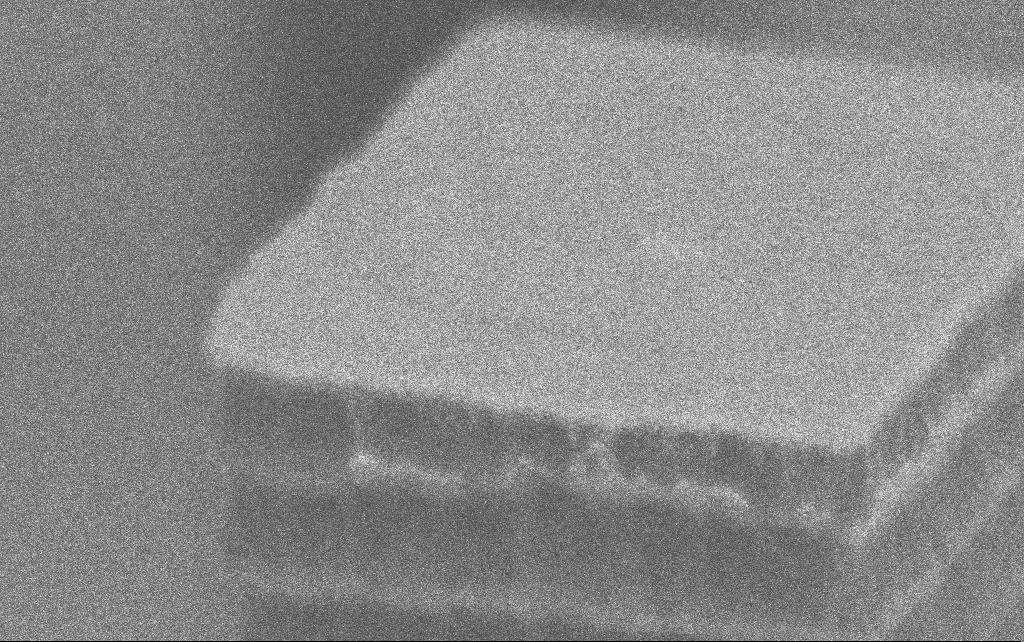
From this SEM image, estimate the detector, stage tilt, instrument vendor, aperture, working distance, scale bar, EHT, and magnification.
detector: InLens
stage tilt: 70°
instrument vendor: Zeiss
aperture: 30 µm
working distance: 3.9 mm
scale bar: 200 nm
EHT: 3 kV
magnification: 130.69 K X